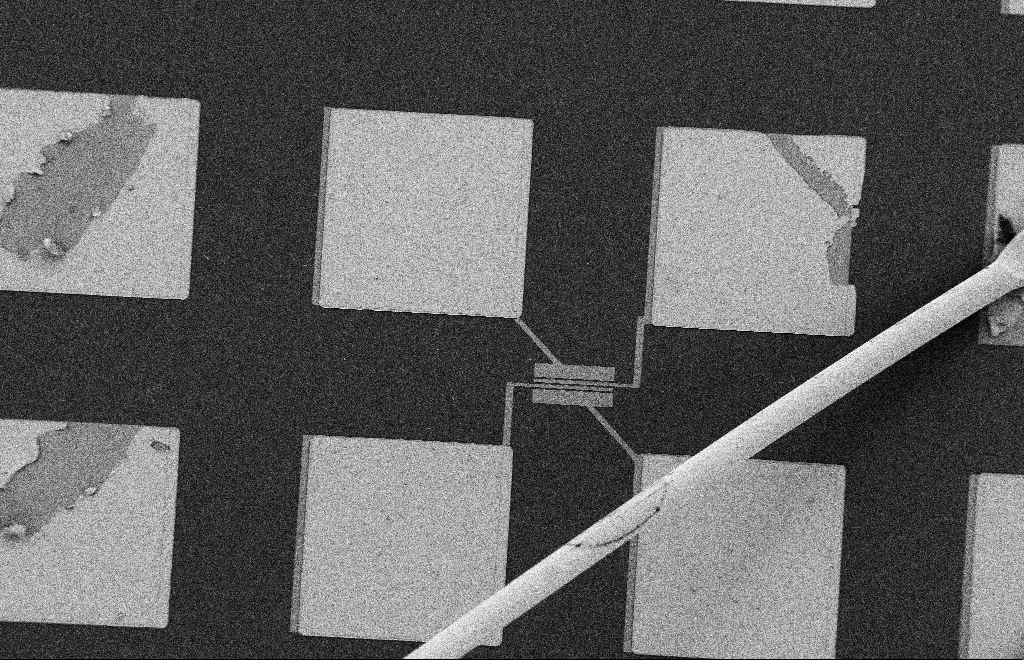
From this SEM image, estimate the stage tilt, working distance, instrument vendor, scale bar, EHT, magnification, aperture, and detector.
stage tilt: -0.3°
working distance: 10 mm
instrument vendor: Zeiss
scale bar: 20000 nm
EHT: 2 kV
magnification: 0.484 K X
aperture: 20 µm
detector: SE2